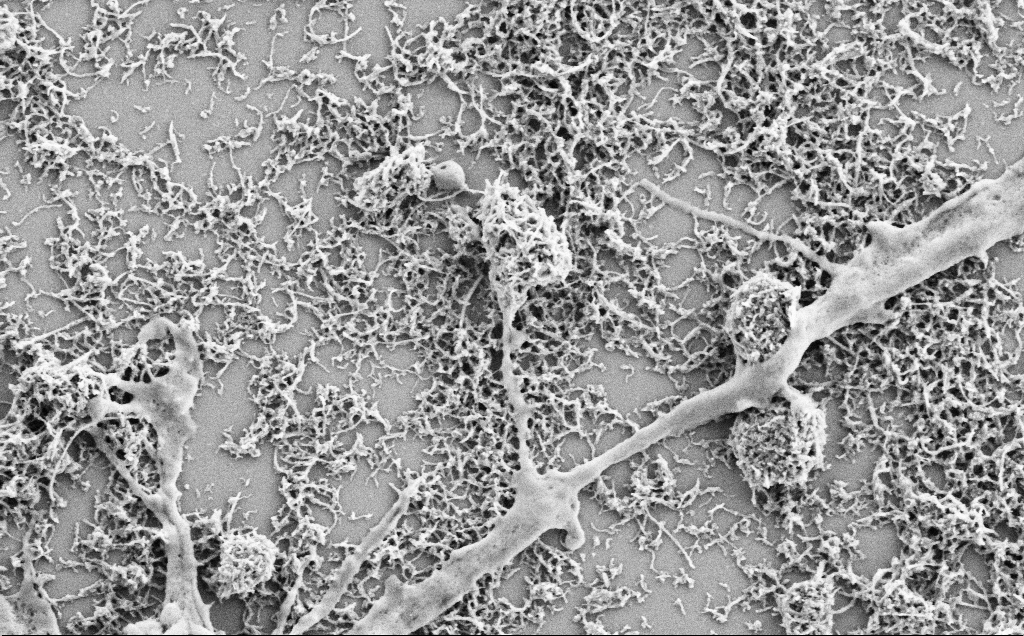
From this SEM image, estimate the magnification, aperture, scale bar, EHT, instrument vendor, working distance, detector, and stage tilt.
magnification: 25 K X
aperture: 30 µm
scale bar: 1000 nm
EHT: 2 kV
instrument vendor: Zeiss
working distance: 7.1 mm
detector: SE2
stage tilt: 0°